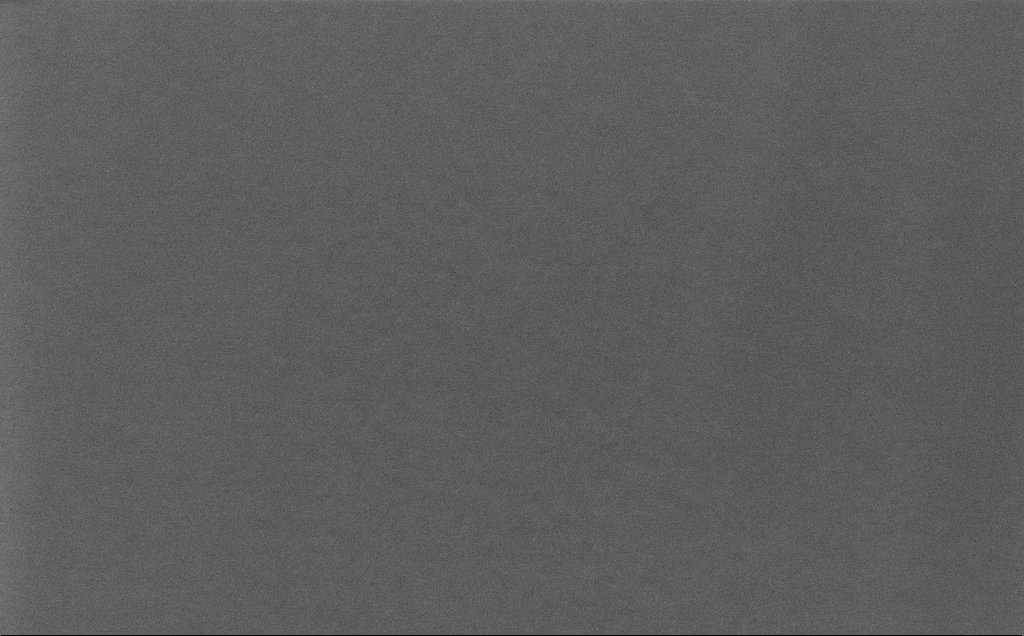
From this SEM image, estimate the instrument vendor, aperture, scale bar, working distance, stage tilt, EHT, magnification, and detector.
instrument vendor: Zeiss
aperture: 30 µm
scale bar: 100 nm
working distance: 12 mm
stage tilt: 0°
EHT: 5 kV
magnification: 458.2 K X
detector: InLens